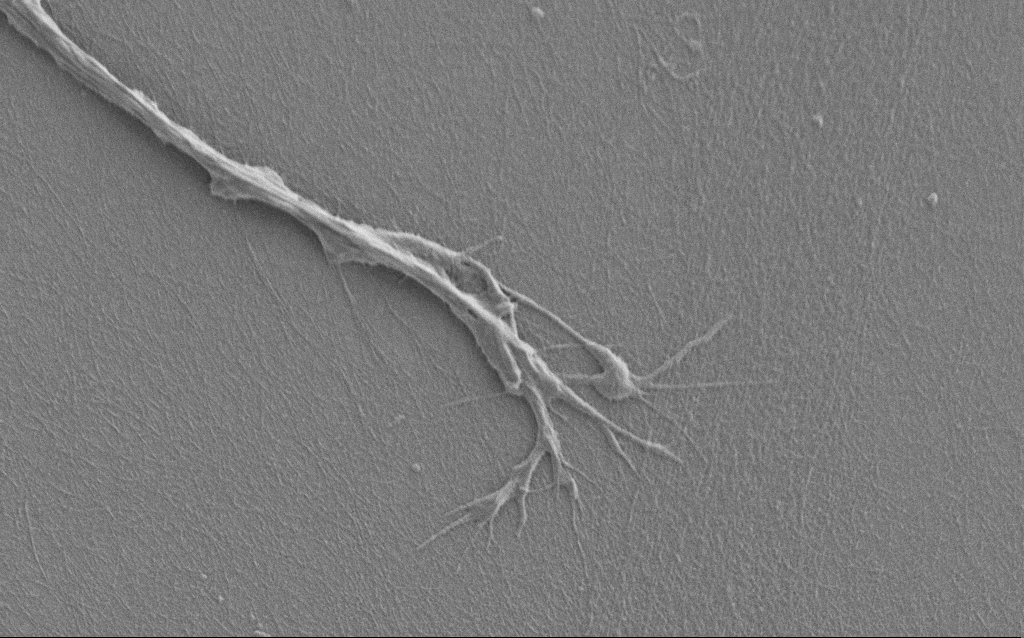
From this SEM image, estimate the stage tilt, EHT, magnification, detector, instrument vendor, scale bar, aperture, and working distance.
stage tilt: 0°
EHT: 1 kV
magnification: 7.5 K X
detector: SE2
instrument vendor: Zeiss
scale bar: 2000 nm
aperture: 30 µm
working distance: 6 mm